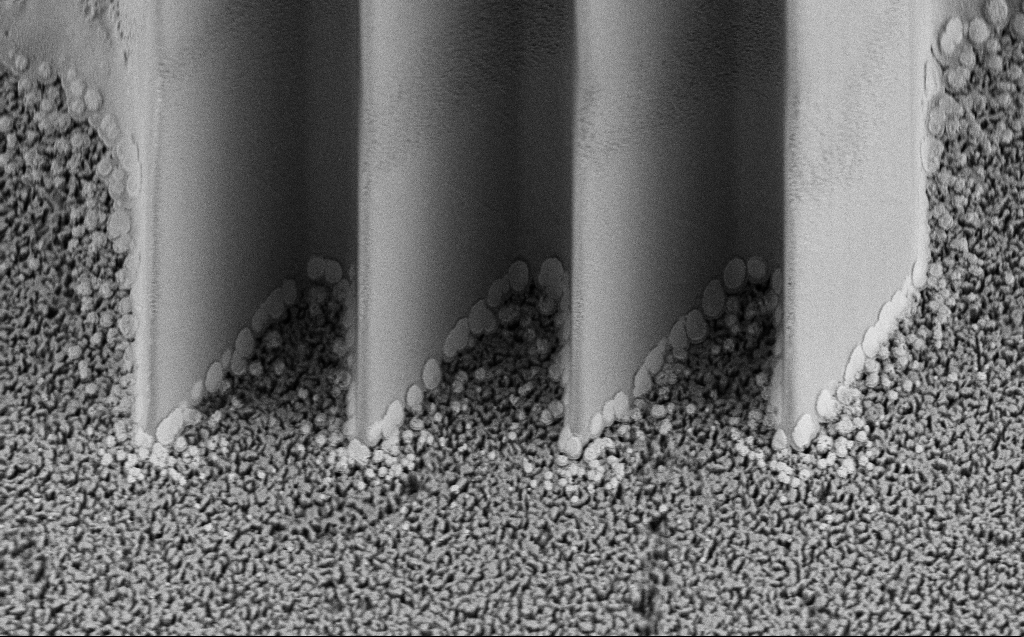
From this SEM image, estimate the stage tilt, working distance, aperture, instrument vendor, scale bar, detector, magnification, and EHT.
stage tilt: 45°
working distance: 5 mm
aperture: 30 µm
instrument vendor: Zeiss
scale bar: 10000 nm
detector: SE2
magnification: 6.84 K X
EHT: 5 kV